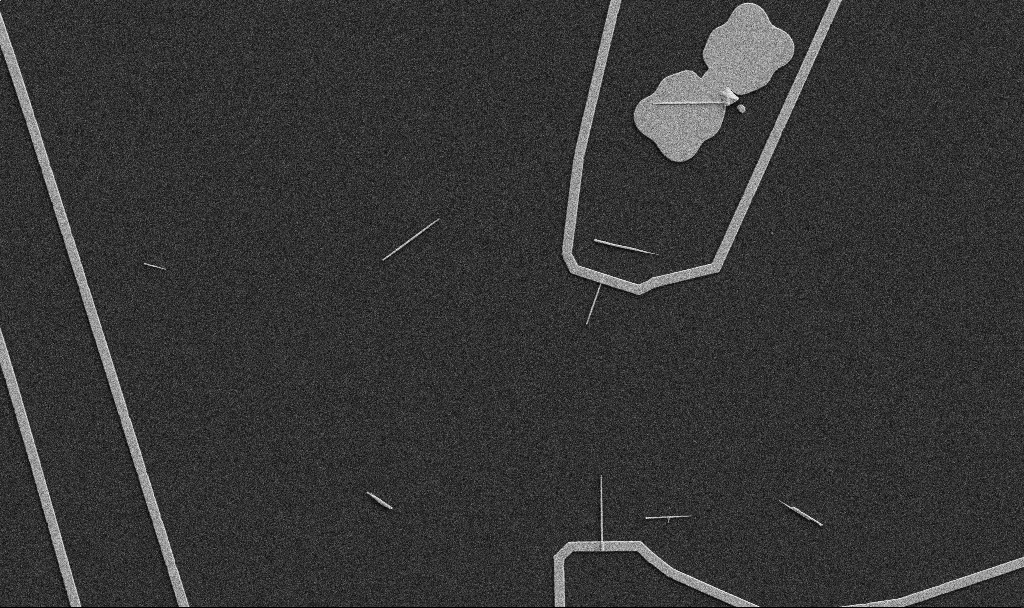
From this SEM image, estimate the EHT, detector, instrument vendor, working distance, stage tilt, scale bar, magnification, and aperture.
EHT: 5 kV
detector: SE2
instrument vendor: Zeiss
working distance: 10.7 mm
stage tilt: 0°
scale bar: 10000 nm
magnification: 5 K X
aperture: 30 µm